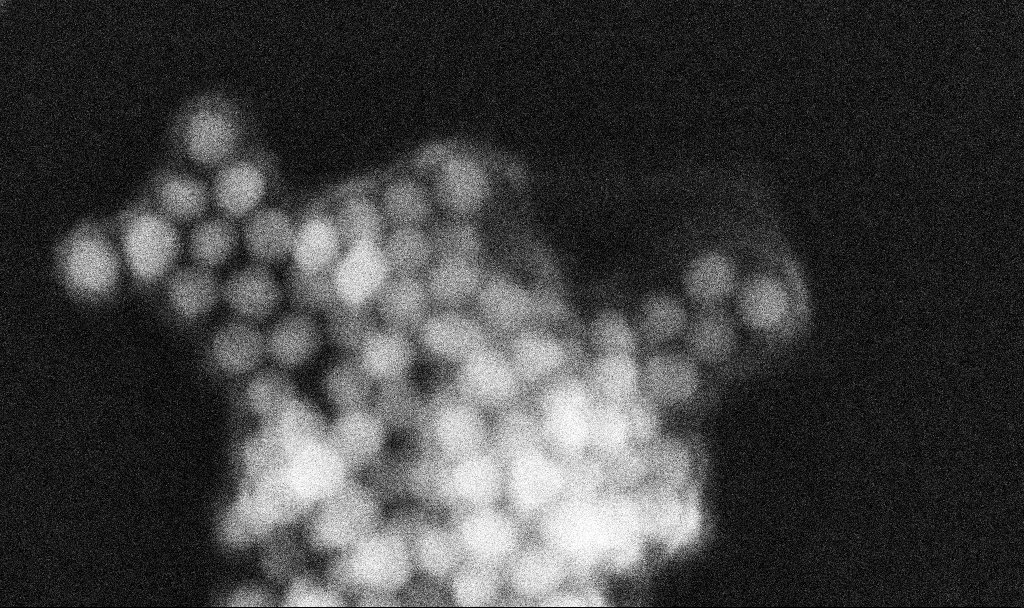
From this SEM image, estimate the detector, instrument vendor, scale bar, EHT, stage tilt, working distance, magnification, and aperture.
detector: InLens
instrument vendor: Zeiss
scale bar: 20 nm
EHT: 10 kV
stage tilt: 0°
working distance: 3.2 mm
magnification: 1012.23 K X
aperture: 30 µm